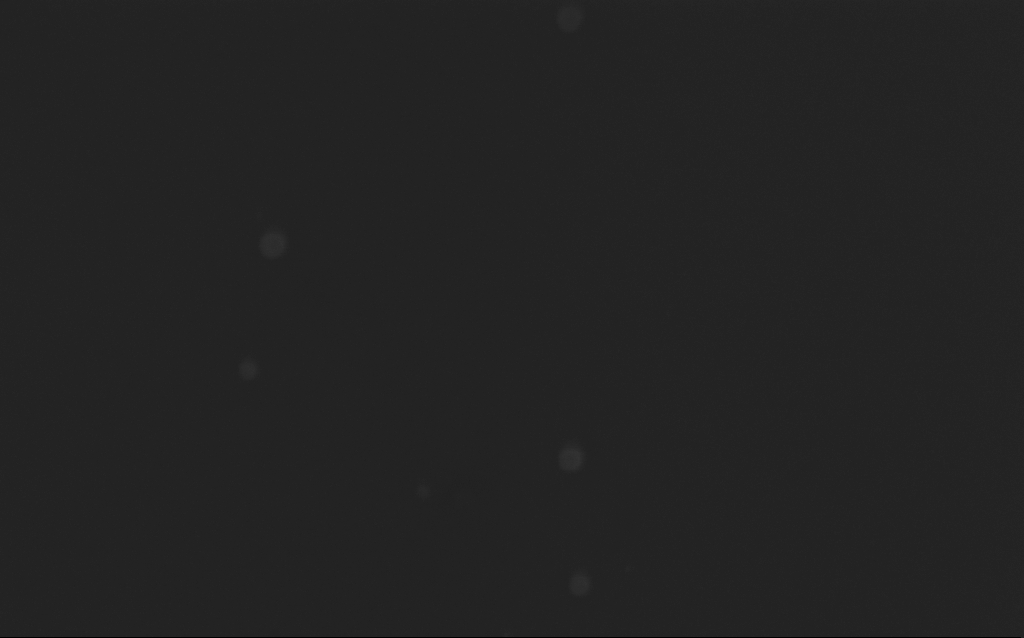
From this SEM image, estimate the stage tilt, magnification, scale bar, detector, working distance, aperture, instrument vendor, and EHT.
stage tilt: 0°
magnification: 283.77 K X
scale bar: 200 nm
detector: InLens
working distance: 4 mm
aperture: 30 µm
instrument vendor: Zeiss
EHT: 5 kV